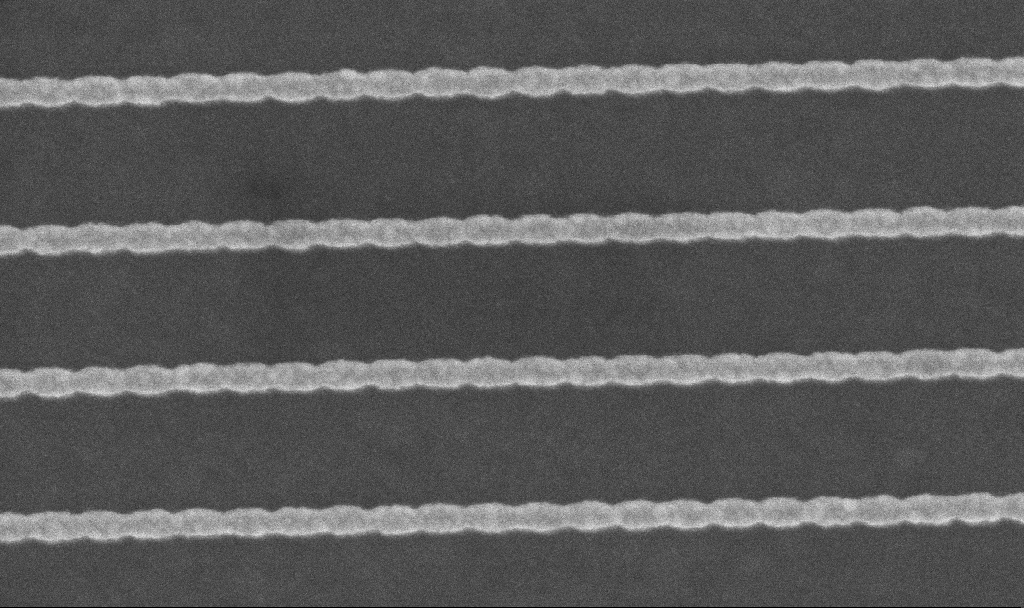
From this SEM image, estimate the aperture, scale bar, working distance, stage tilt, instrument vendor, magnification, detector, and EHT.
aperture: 30 µm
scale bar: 200 nm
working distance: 6 mm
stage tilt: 0°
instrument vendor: Zeiss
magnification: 178.05 K X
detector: InLens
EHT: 5 kV